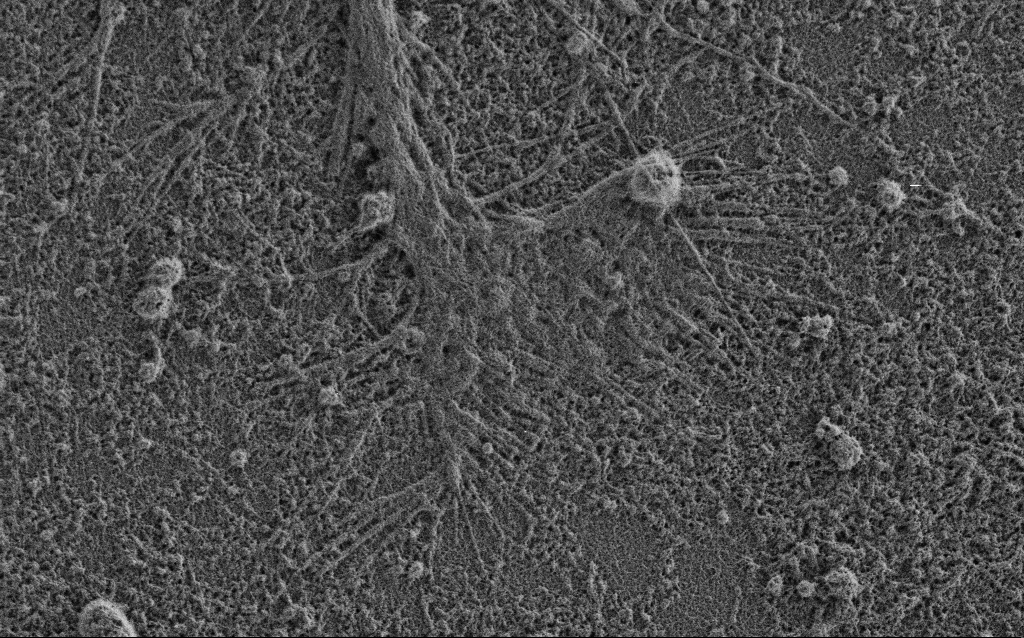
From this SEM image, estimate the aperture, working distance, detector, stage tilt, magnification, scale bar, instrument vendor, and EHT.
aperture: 30 µm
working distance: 3.4 mm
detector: SE2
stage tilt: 0°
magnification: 10 K X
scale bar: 2000 nm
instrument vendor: Zeiss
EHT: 0.9 kV